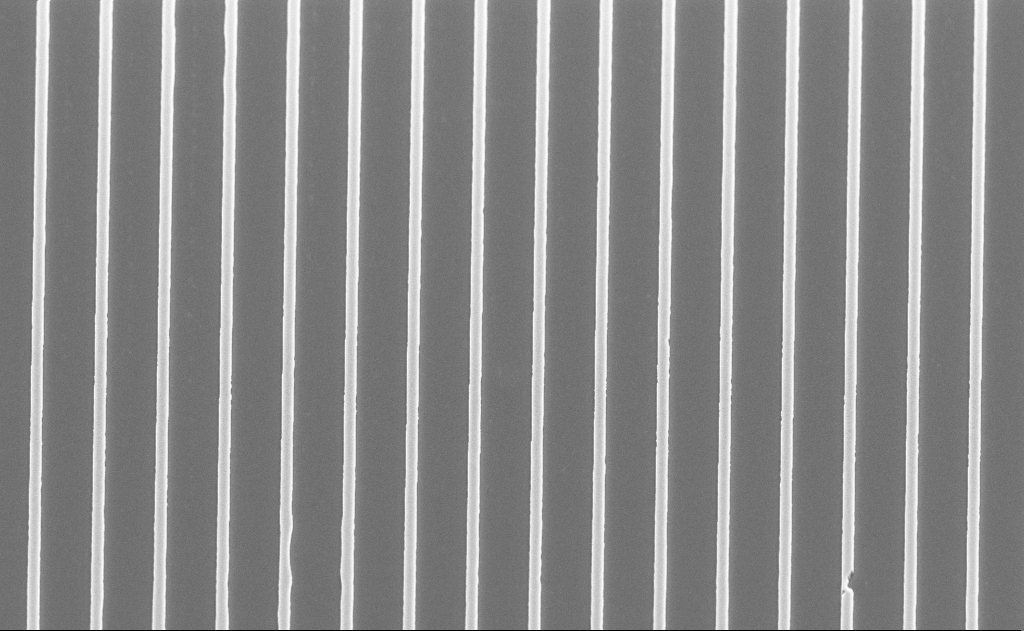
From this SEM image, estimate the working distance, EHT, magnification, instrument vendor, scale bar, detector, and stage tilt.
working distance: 13 mm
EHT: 5 kV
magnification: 5.72 K X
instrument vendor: Zeiss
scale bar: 10000 nm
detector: SE2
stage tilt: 55°